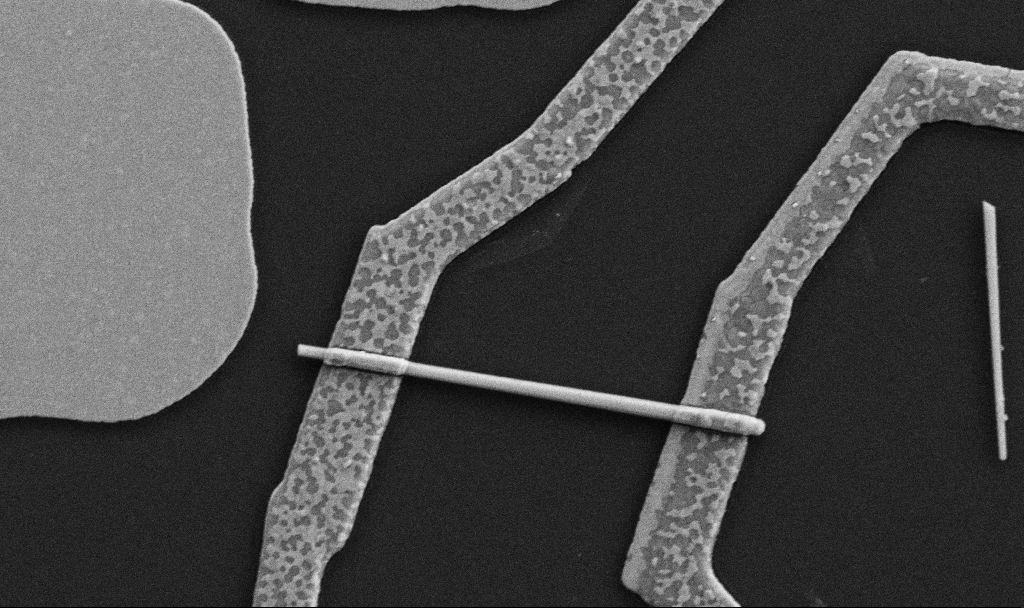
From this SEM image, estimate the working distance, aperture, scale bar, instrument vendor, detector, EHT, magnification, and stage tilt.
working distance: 8.7 mm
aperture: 30 µm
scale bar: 1000 nm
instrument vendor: Zeiss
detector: SE2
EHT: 5 kV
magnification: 30 K X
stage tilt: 0°